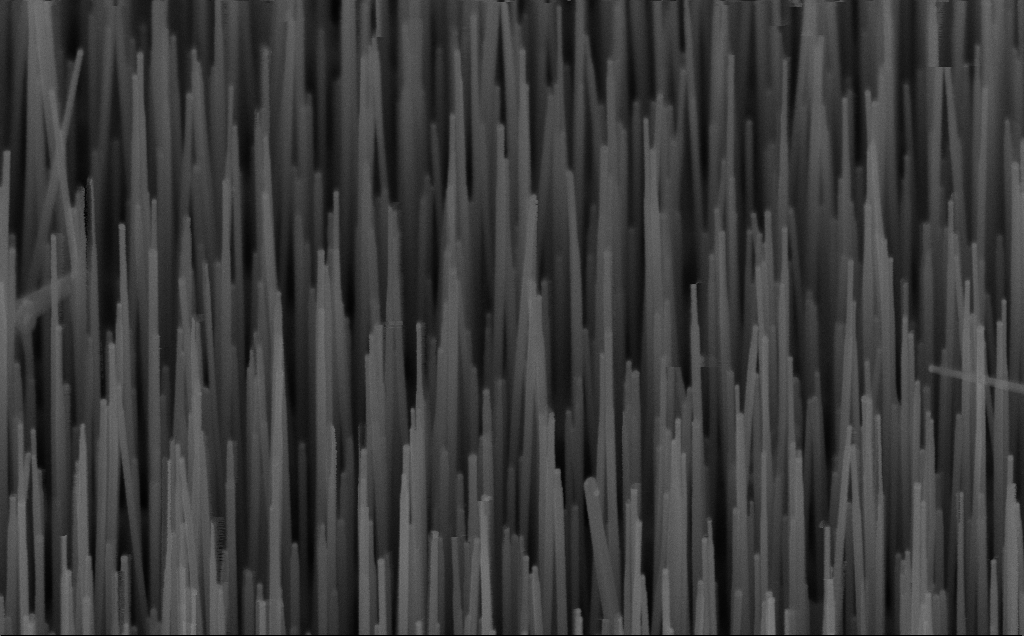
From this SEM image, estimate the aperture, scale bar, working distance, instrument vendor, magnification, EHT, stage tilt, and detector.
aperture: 30 µm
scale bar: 200 nm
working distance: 7 mm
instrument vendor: Zeiss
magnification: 100 K X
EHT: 10 kV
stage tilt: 45°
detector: InLens